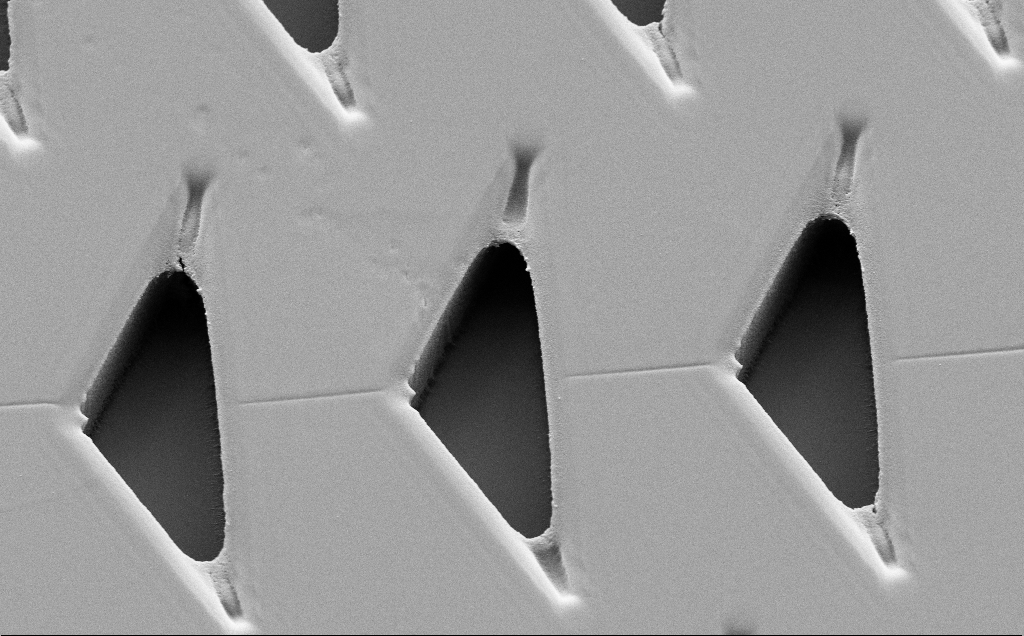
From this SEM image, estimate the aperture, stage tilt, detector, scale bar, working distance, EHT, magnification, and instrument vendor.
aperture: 30 µm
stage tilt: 30°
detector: SE2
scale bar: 10000 nm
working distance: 9 mm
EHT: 5 kV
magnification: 5.03 K X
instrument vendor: Zeiss